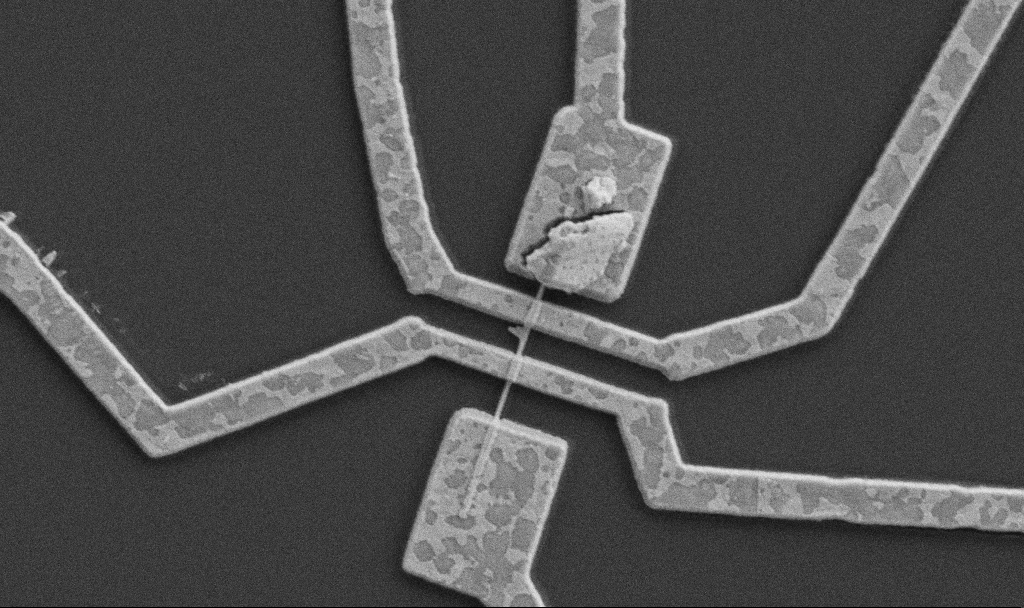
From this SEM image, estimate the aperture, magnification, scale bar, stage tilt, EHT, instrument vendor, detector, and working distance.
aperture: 30 µm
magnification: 20 K X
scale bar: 1000 nm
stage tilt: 0°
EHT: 5 kV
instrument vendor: Zeiss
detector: SE2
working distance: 8.7 mm